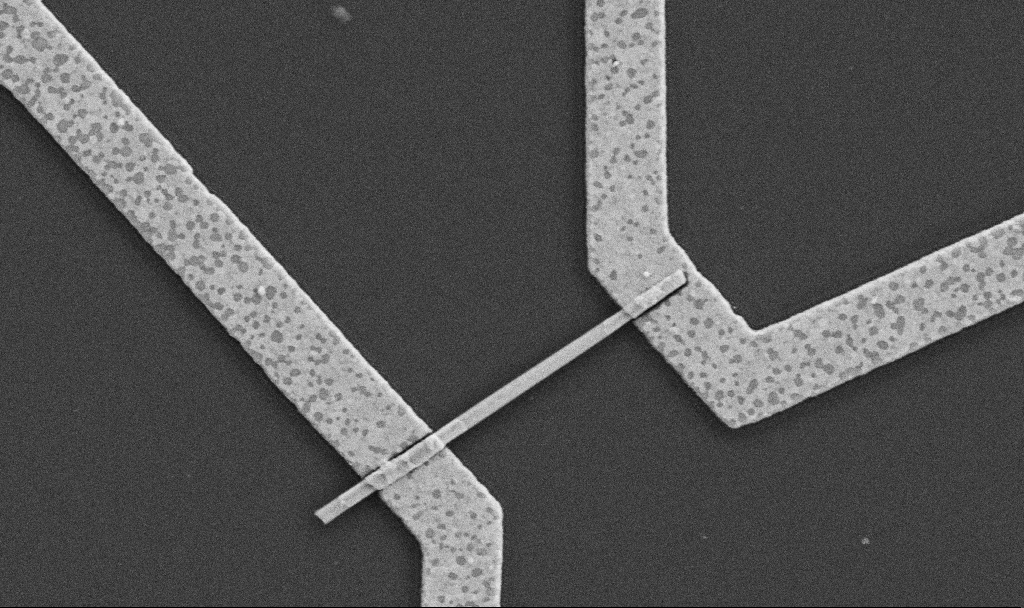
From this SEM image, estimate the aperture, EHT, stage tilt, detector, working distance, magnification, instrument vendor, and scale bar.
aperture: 30 µm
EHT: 5 kV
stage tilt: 0°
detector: SE2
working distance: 8.7 mm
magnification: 30 K X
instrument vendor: Zeiss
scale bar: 1000 nm